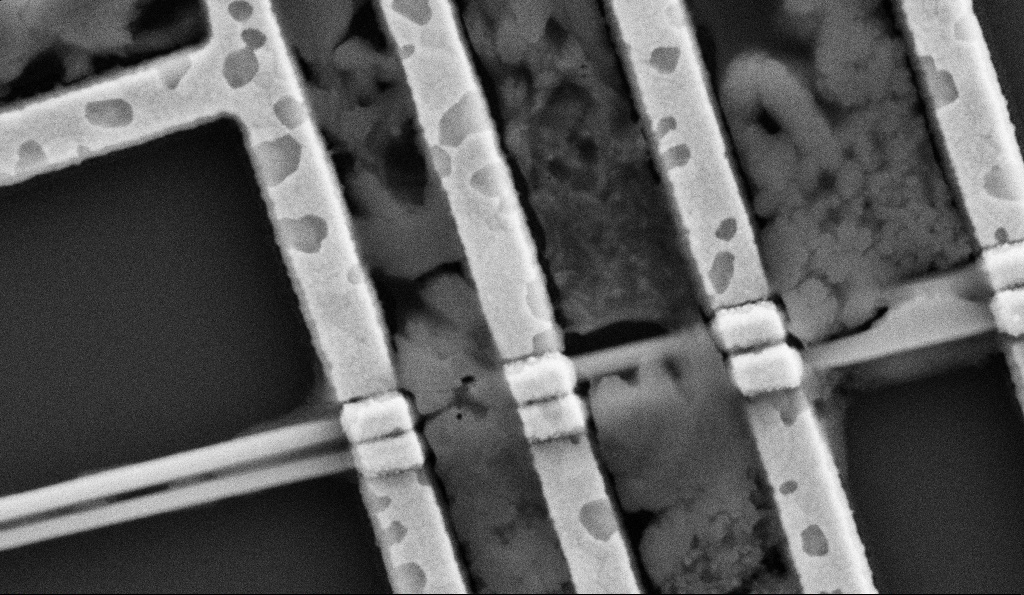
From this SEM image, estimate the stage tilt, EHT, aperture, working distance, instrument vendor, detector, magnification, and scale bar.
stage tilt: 0°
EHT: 5 kV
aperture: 30 µm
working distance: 6.7 mm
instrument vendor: Zeiss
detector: SE2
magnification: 100 K X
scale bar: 200 nm